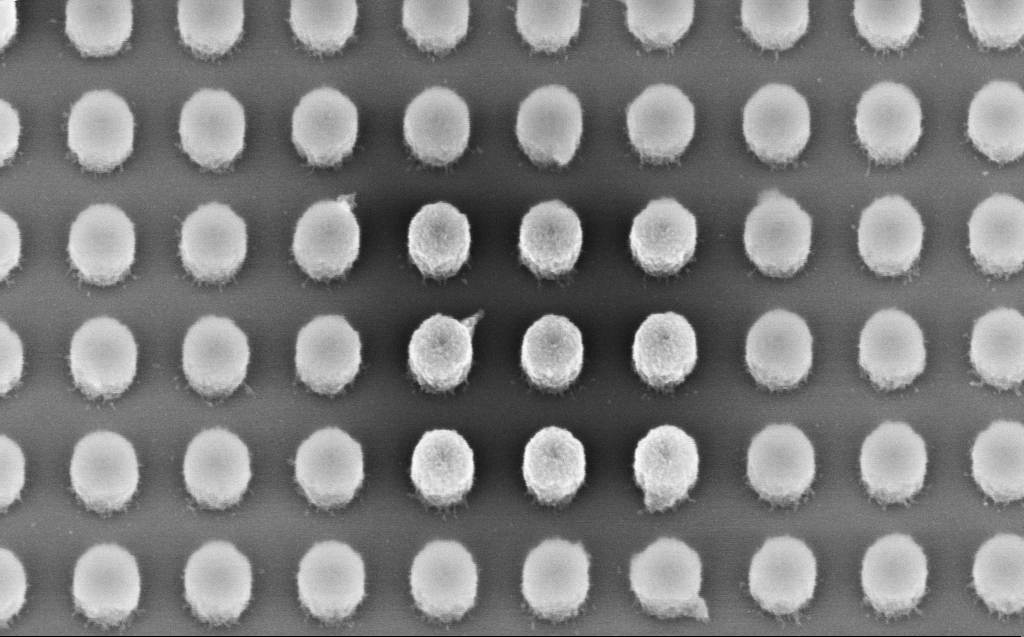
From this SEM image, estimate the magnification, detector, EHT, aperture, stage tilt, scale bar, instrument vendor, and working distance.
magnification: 103.97 K X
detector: InLens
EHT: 3 kV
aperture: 30 µm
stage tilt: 30°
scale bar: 200 nm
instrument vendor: Zeiss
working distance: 6 mm